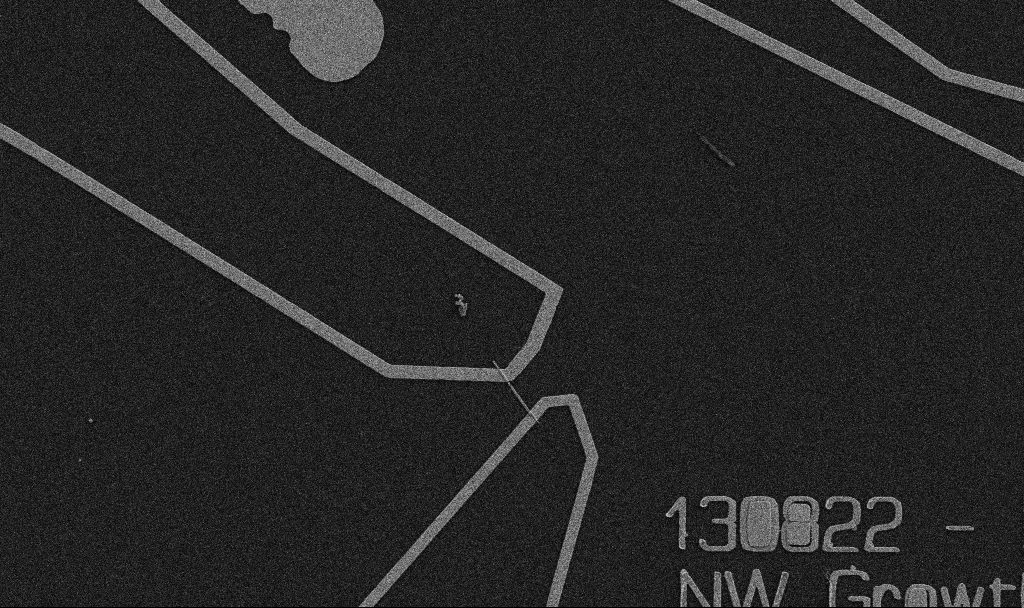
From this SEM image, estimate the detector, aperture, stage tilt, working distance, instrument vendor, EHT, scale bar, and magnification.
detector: SE2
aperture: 30 µm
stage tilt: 0°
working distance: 10.7 mm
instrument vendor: Zeiss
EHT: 5 kV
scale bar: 10000 nm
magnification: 5 K X